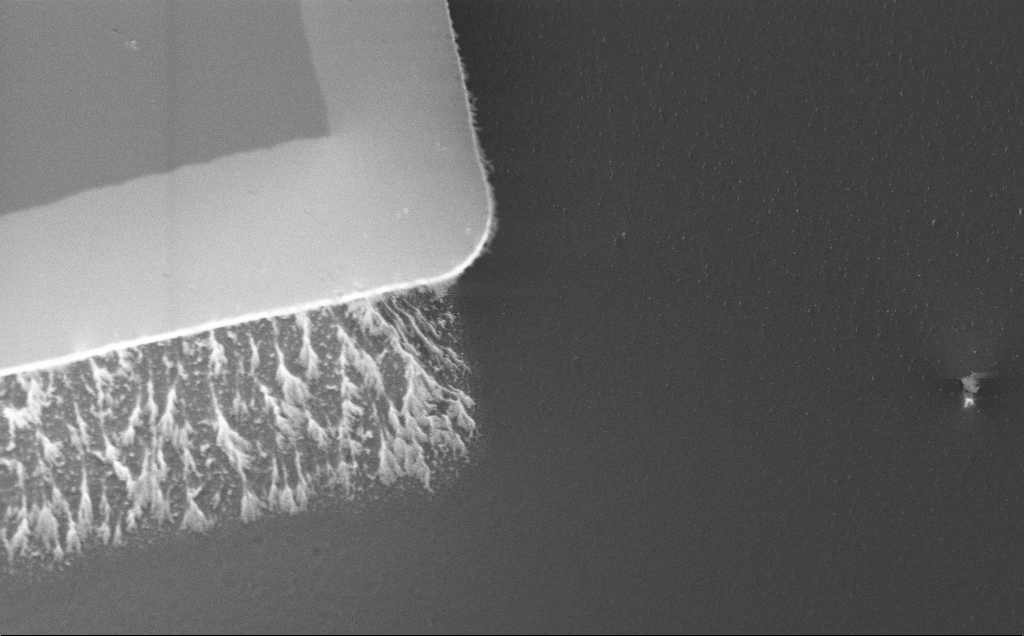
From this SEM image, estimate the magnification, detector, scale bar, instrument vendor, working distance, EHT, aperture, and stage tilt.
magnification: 7.81 K X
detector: InLens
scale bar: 2000 nm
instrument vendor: Zeiss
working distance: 8 mm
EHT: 5 kV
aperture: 30 µm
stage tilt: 45°